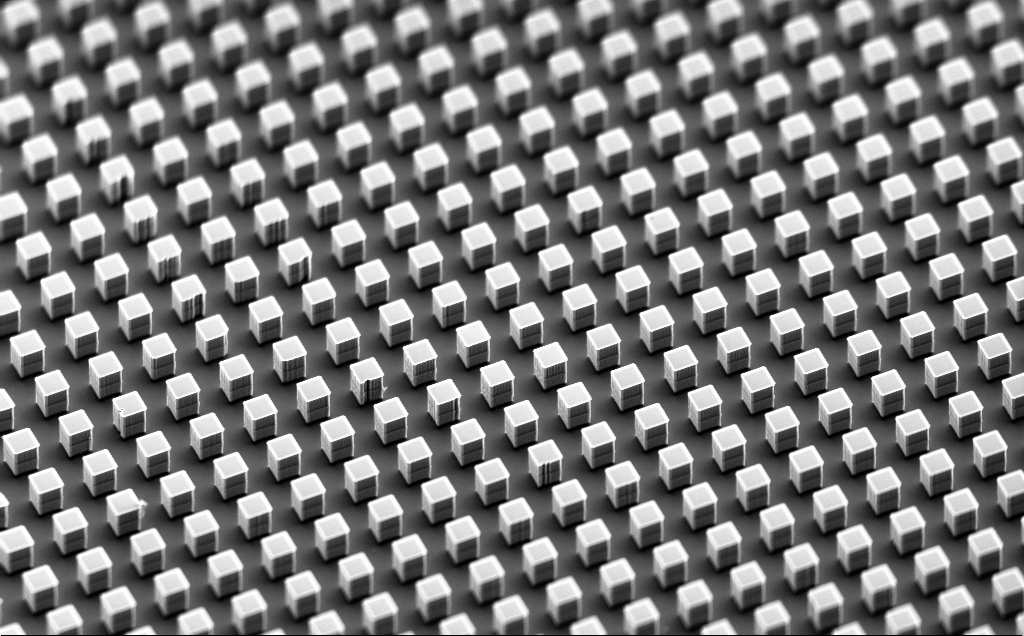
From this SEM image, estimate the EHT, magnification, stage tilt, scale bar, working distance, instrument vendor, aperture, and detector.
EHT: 10 kV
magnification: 1.07 K X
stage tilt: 48.7°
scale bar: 20000 nm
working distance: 11 mm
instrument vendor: Zeiss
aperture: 120 µm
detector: SE2